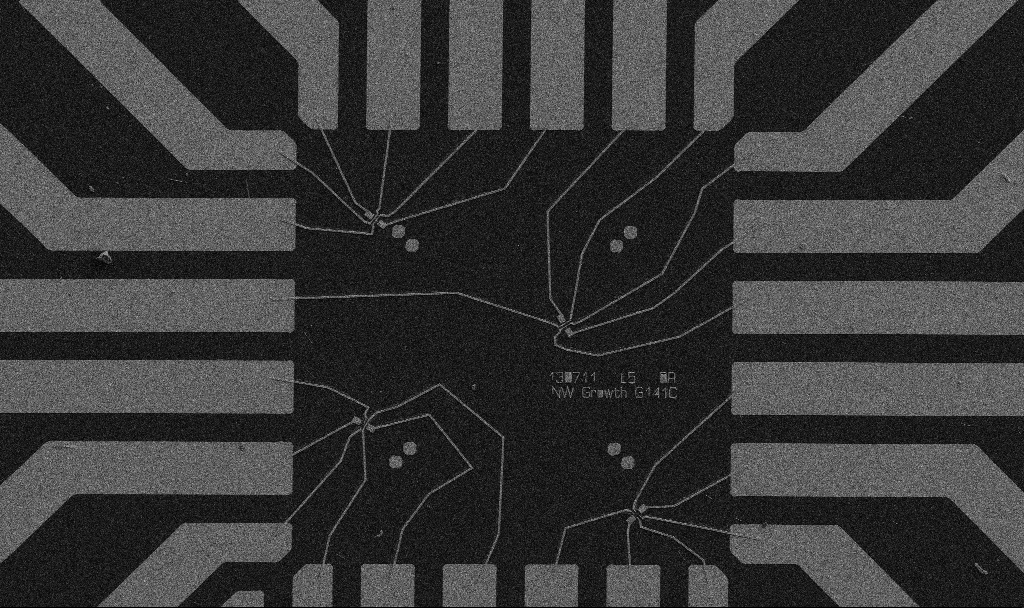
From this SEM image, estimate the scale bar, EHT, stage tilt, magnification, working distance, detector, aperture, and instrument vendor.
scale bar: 20000 nm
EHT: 5 kV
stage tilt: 0°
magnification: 1 K X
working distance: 10.7 mm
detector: SE2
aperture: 30 µm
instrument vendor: Zeiss